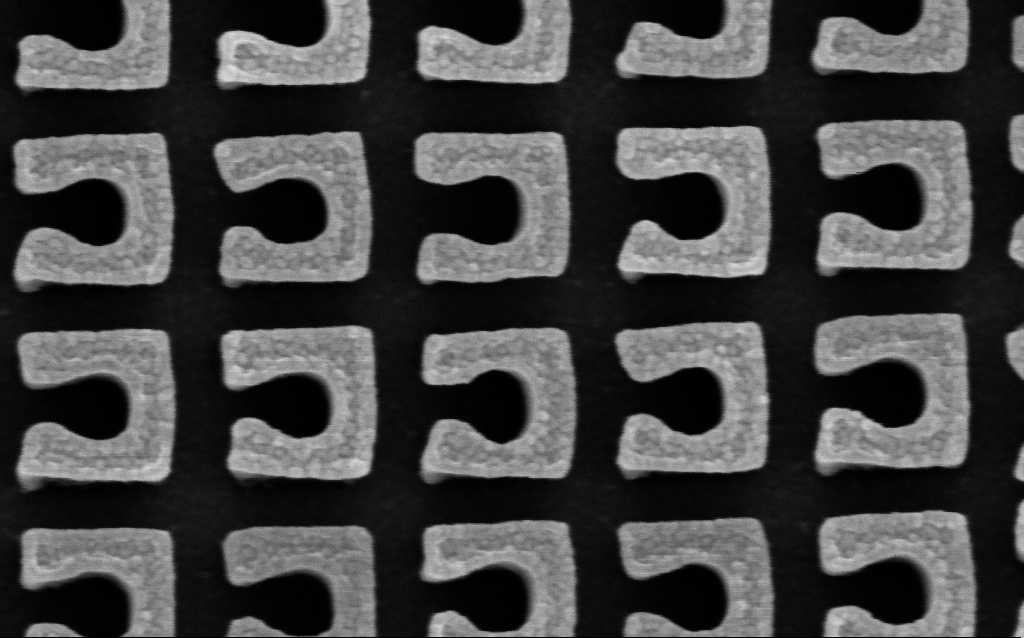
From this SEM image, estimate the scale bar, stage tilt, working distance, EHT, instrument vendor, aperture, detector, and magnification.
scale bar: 200 nm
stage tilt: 0°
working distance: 4.6 mm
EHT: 3 kV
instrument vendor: Zeiss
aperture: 30 µm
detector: InLens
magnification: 159.36 K X